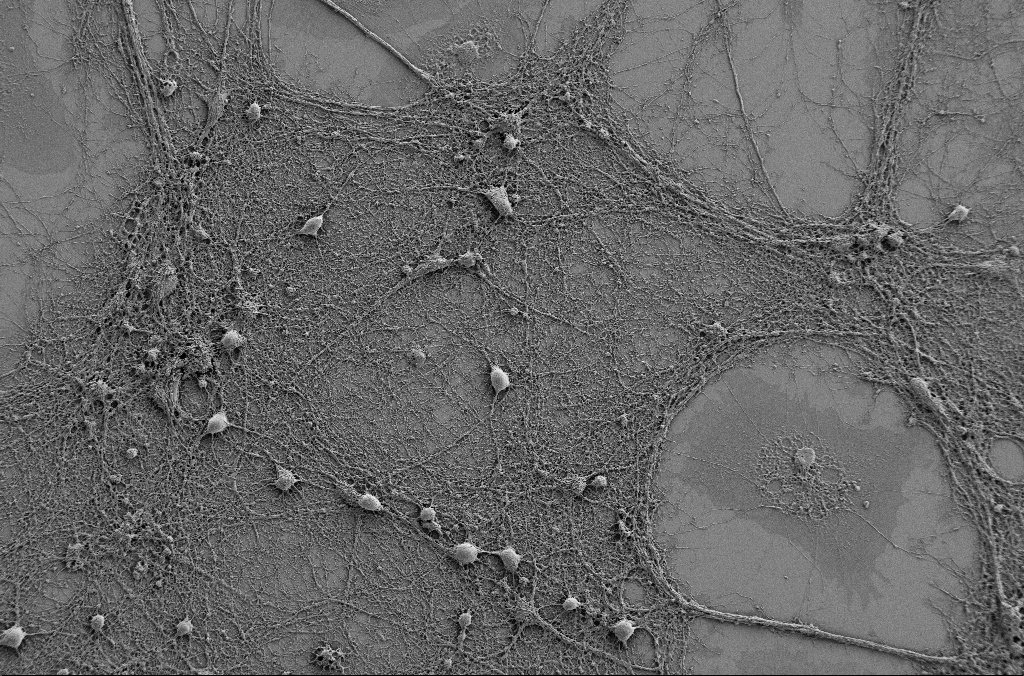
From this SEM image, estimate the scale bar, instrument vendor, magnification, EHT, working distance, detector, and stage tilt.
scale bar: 20000 nm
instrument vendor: Zeiss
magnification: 1 K X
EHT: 1 kV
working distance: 4.1 mm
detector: SE2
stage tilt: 0°